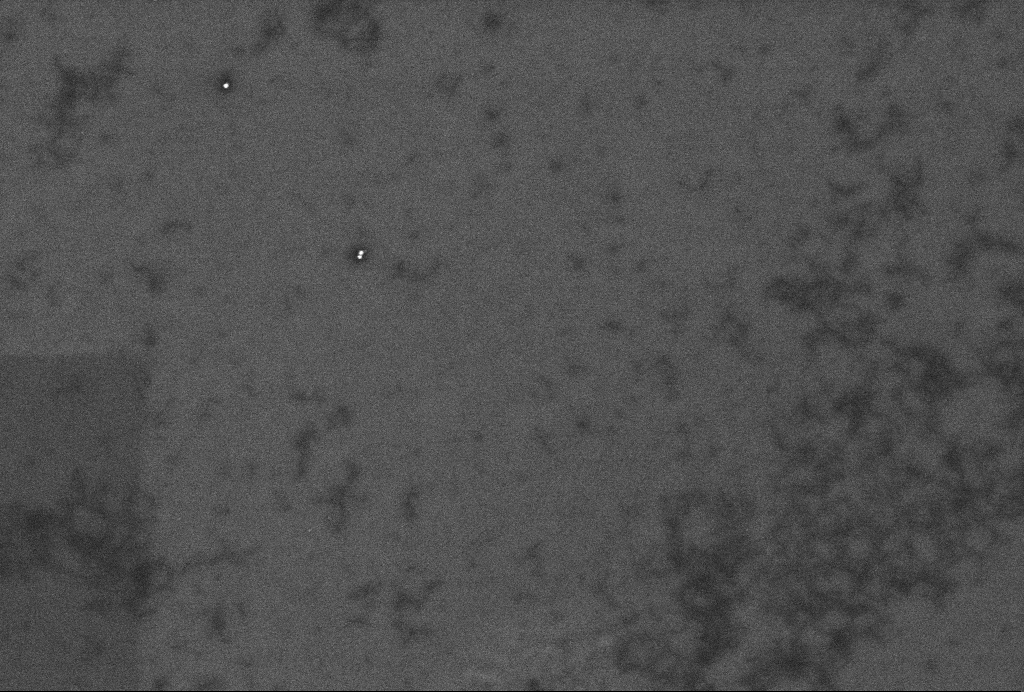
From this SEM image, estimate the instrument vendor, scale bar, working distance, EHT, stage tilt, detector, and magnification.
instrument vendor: Zeiss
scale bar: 200 nm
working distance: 3.3 mm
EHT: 2 kV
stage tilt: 0°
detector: InLens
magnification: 64.15 K X